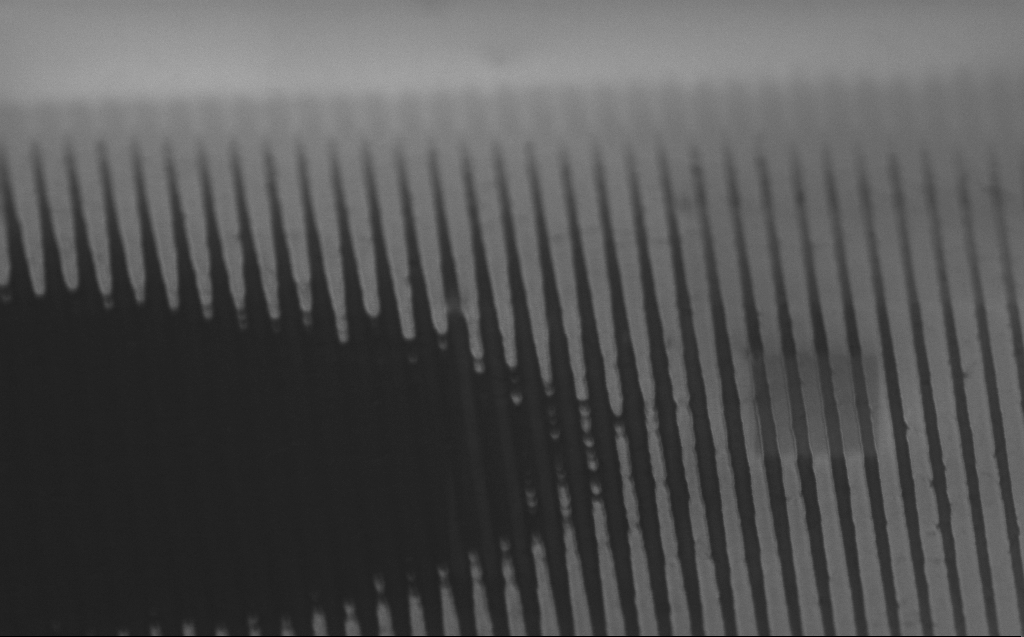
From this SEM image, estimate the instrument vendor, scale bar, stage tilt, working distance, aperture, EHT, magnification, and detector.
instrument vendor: Zeiss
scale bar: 2000 nm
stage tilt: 60.7°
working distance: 3 mm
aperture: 30 µm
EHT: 1 kV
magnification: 24.56 K X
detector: InLens